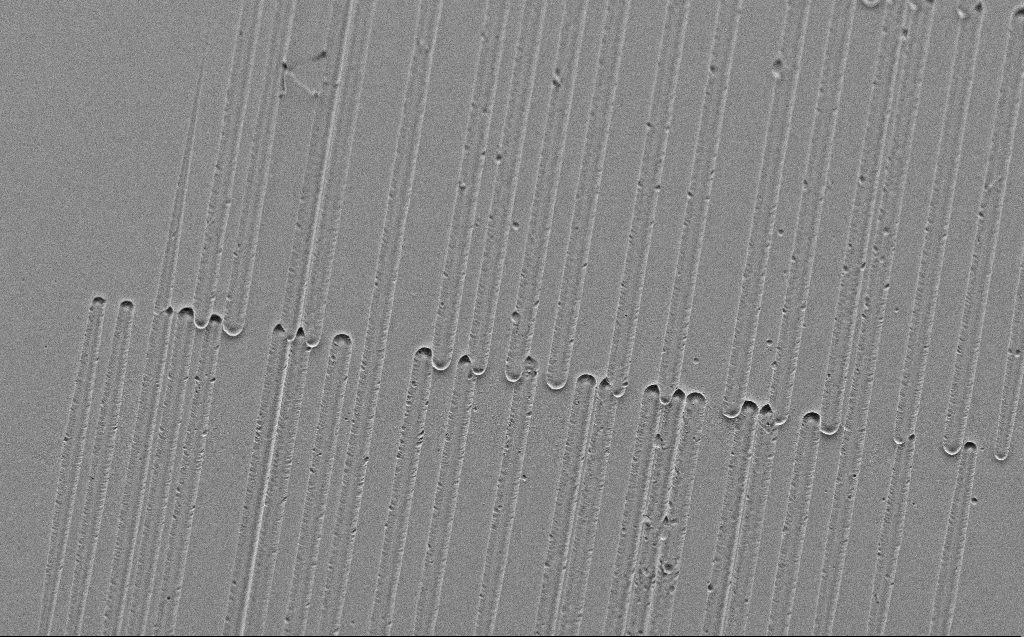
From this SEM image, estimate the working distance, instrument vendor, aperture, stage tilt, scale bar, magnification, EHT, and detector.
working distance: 5 mm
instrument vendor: Zeiss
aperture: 30 µm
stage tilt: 45°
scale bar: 10000 nm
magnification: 1.64 K X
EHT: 3 kV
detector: SE2